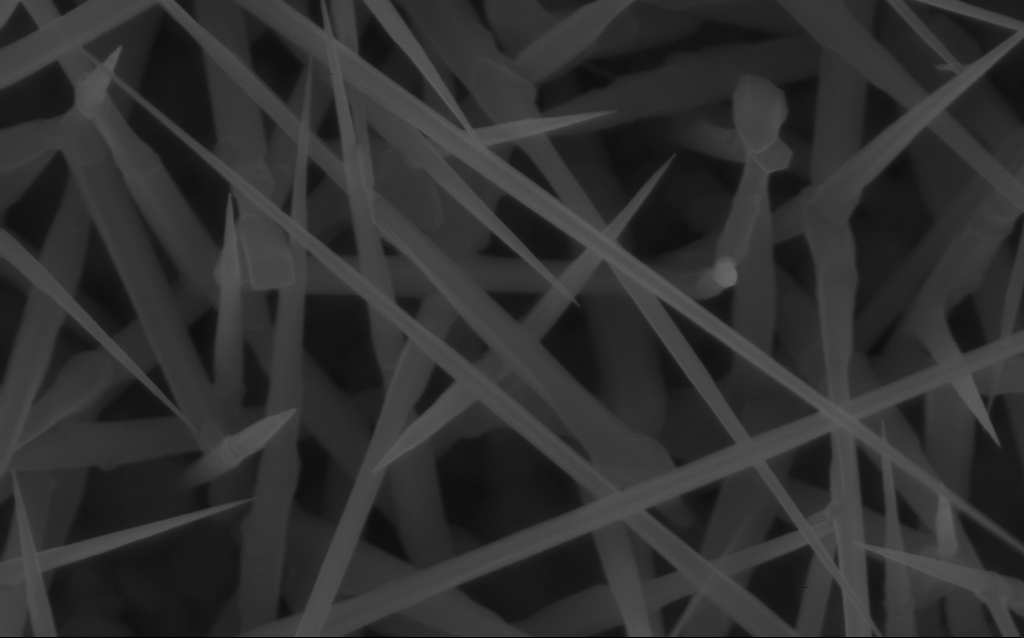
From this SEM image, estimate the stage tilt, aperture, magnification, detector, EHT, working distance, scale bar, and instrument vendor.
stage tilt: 0°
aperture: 30 µm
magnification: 80 K X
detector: InLens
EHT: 10 kV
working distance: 7 mm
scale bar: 200 nm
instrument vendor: Zeiss